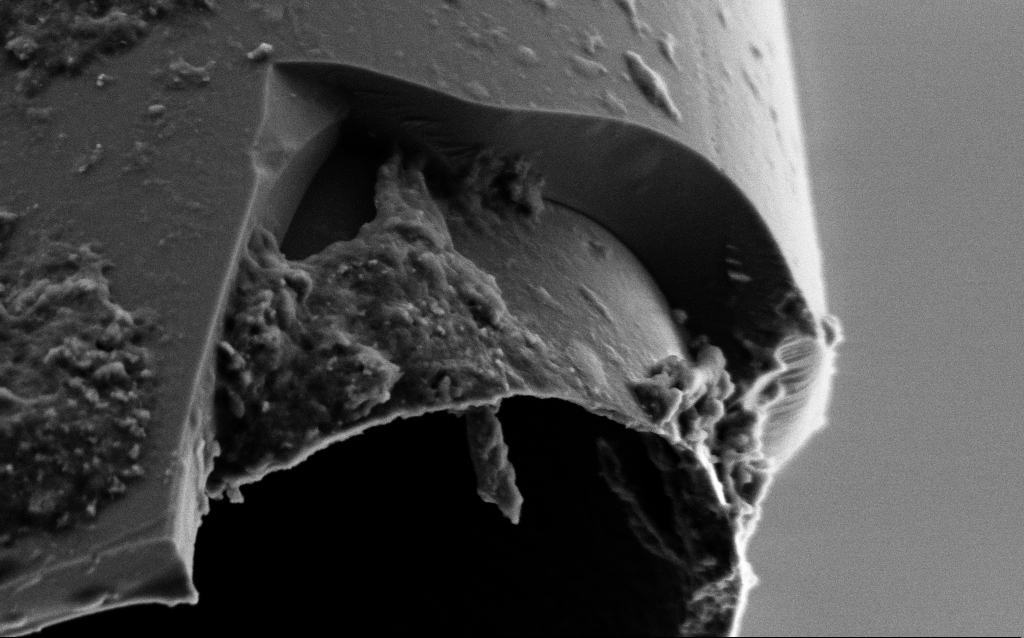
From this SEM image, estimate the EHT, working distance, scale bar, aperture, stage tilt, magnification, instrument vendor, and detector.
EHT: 2 kV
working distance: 6 mm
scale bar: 1000 nm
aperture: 30 µm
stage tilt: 45°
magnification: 50 K X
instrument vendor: Zeiss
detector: SE2